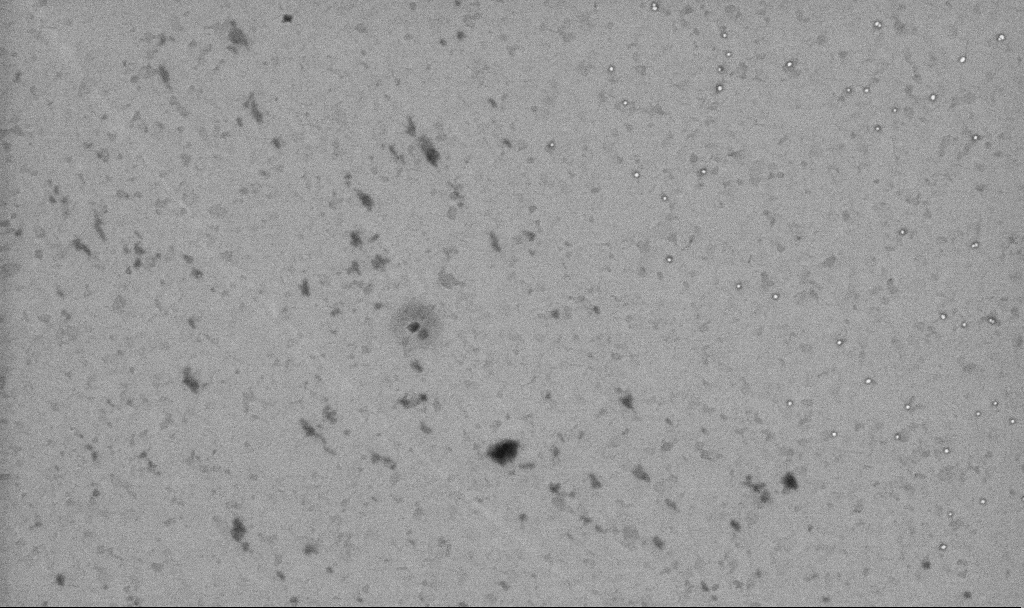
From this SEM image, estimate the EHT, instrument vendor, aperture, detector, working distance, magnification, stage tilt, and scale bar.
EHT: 10 kV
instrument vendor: Zeiss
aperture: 30 µm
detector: InLens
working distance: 3.3 mm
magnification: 50.38 K X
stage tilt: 0°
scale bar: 1000 nm